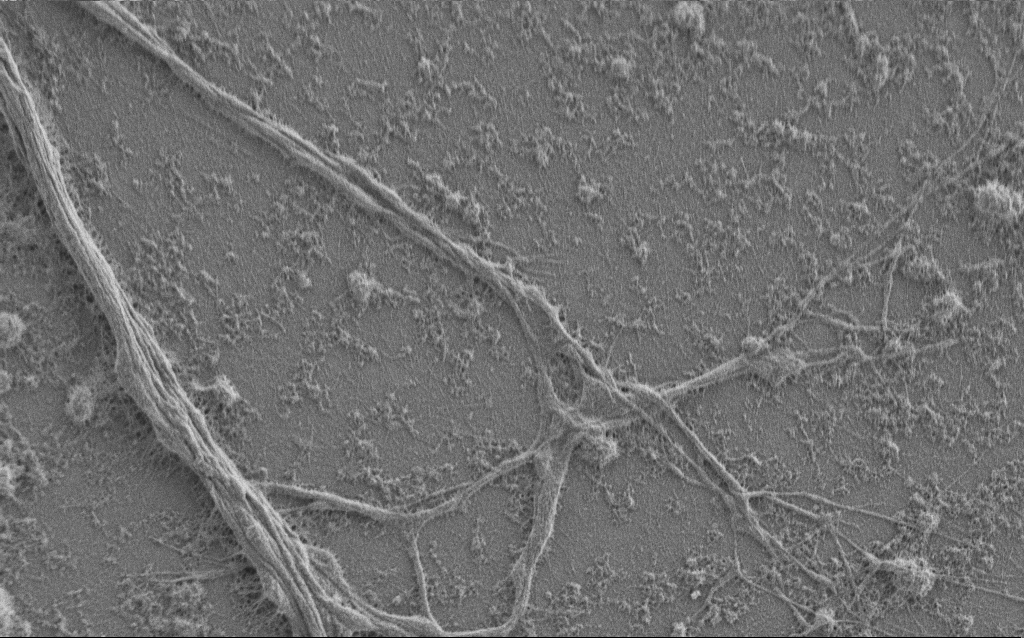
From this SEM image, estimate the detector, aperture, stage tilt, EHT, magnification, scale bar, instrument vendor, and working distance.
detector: SE2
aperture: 30 µm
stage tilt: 0°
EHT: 1 kV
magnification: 7.5 K X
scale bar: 2000 nm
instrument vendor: Zeiss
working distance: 6 mm